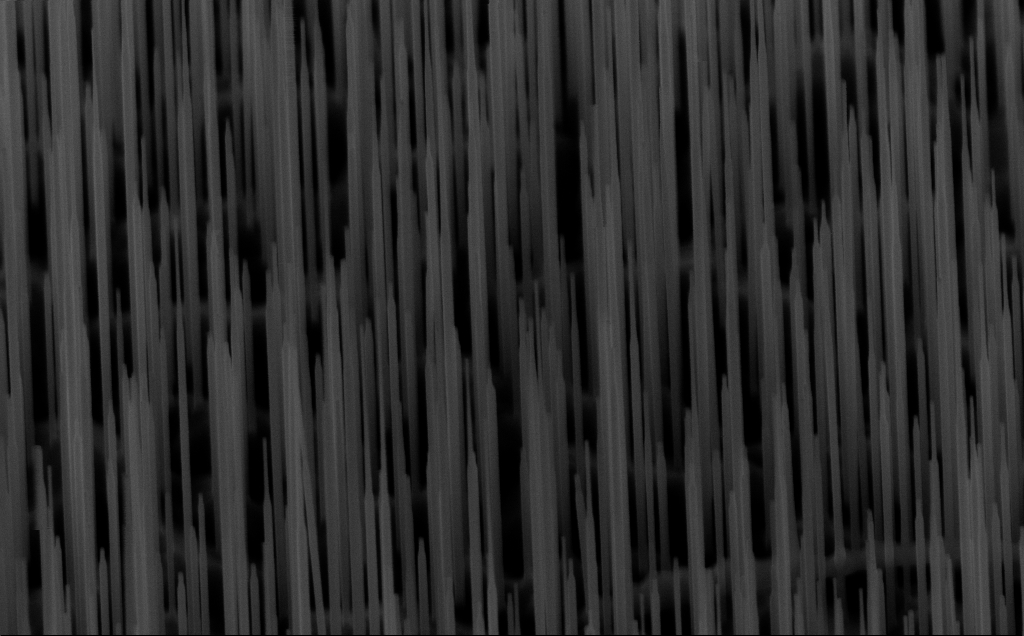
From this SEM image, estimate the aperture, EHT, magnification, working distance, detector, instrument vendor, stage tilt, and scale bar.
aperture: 30 µm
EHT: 10 kV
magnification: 40 K X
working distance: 6 mm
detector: InLens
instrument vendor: Zeiss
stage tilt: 45°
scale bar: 1000 nm